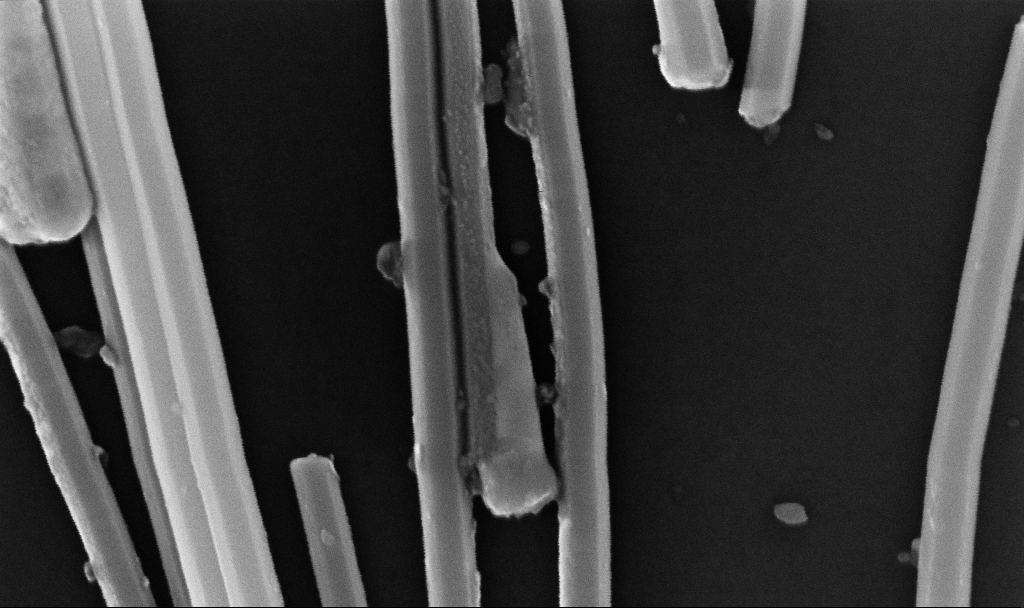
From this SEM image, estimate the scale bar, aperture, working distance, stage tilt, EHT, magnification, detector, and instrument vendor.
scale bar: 200 nm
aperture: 30 µm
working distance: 6.7 mm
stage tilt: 0°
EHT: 10 kV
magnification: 182.13 K X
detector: InLens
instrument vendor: Zeiss